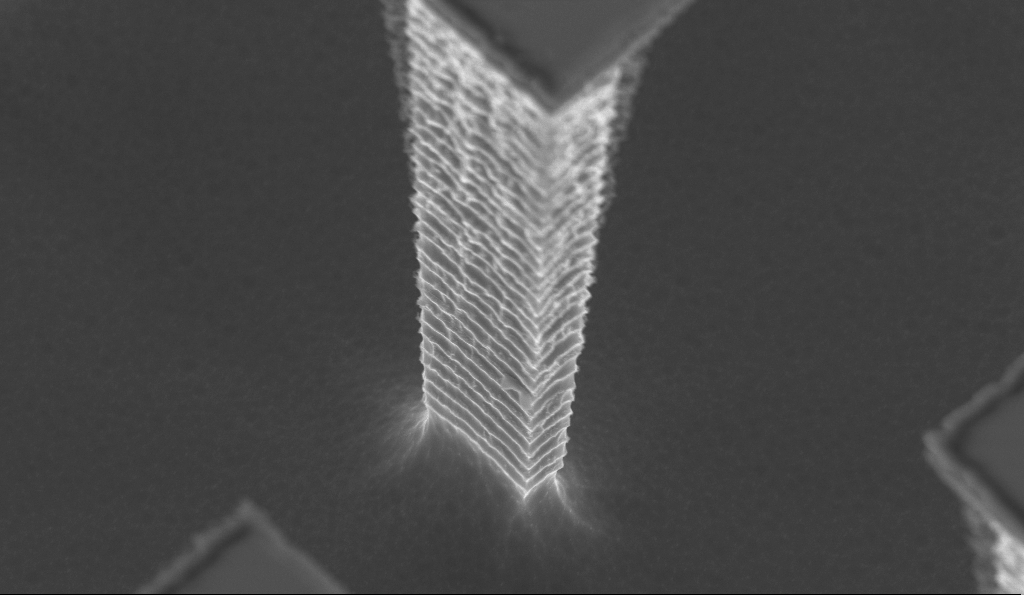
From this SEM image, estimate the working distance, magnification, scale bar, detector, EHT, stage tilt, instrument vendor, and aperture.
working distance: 5.5 mm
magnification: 25.76 K X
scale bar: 2000 nm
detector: InLens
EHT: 5 kV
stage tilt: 30°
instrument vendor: Zeiss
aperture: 30 µm